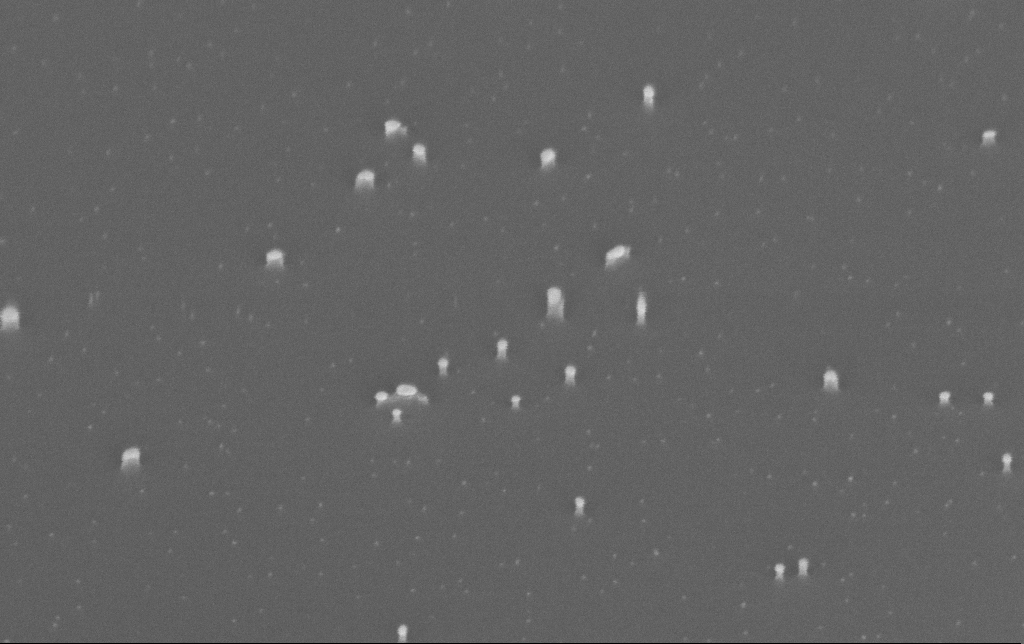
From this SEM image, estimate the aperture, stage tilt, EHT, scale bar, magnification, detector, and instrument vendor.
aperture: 30 µm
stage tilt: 45°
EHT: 10 kV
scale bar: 200 nm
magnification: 200 K X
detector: InLens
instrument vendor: Zeiss